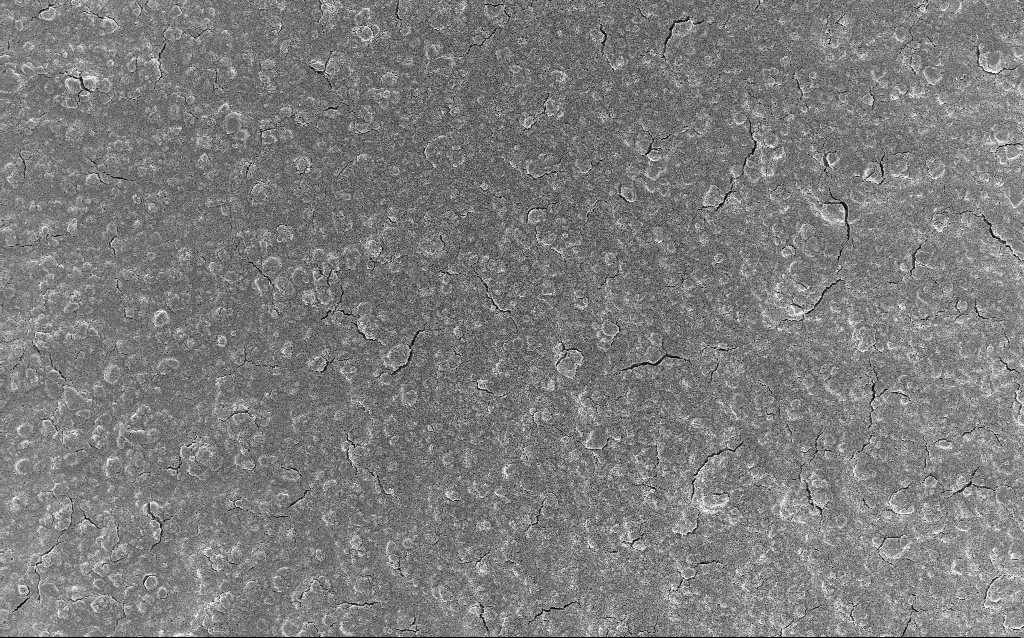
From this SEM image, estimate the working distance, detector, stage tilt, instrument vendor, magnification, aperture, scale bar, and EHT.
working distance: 2.9 mm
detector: InLens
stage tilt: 0°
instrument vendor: Zeiss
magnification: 0.77 K X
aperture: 30 µm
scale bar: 20000 nm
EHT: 5 kV